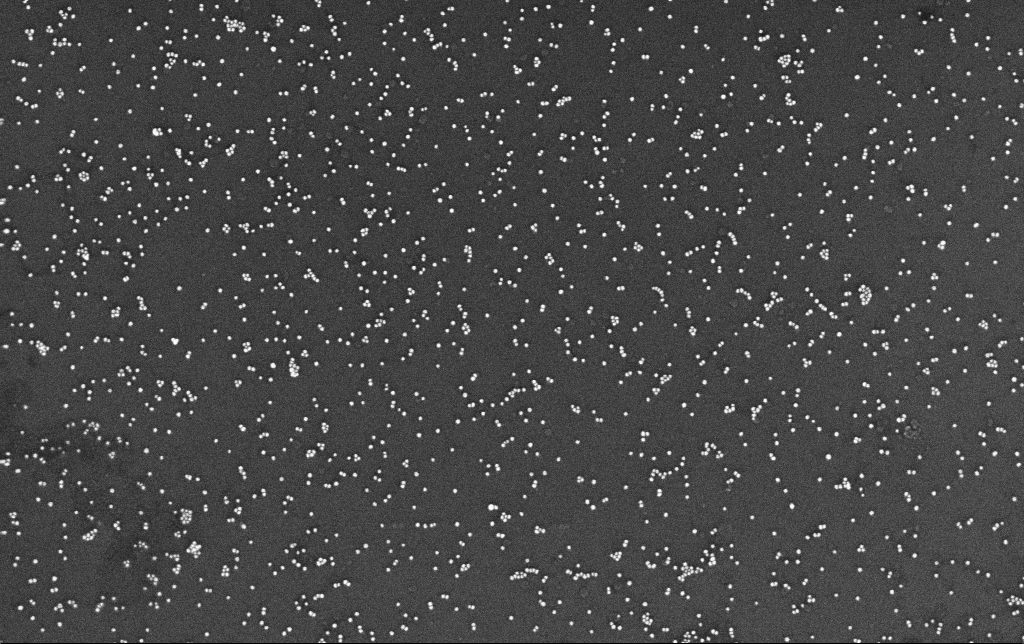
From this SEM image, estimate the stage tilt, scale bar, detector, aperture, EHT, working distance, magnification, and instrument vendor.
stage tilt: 0°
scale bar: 200 nm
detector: InLens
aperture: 30 µm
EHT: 10 kV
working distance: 3.4 mm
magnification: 100 K X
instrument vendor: Zeiss